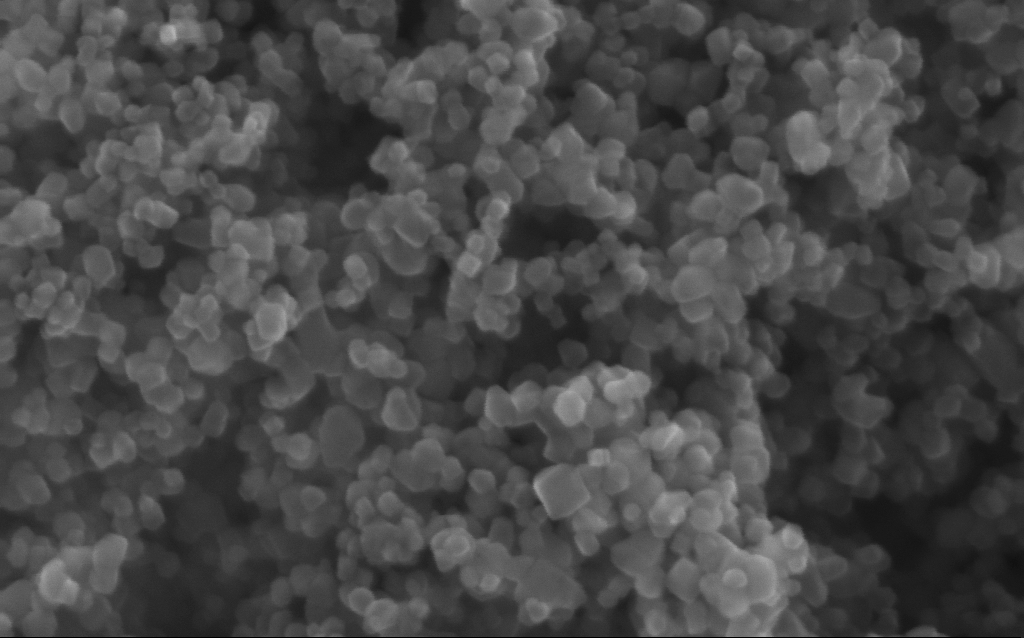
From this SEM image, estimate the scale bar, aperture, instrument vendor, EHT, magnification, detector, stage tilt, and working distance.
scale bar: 100 nm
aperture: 30 µm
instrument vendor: Zeiss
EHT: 10 kV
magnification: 416 K X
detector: InLens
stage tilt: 0°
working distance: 2.7 mm